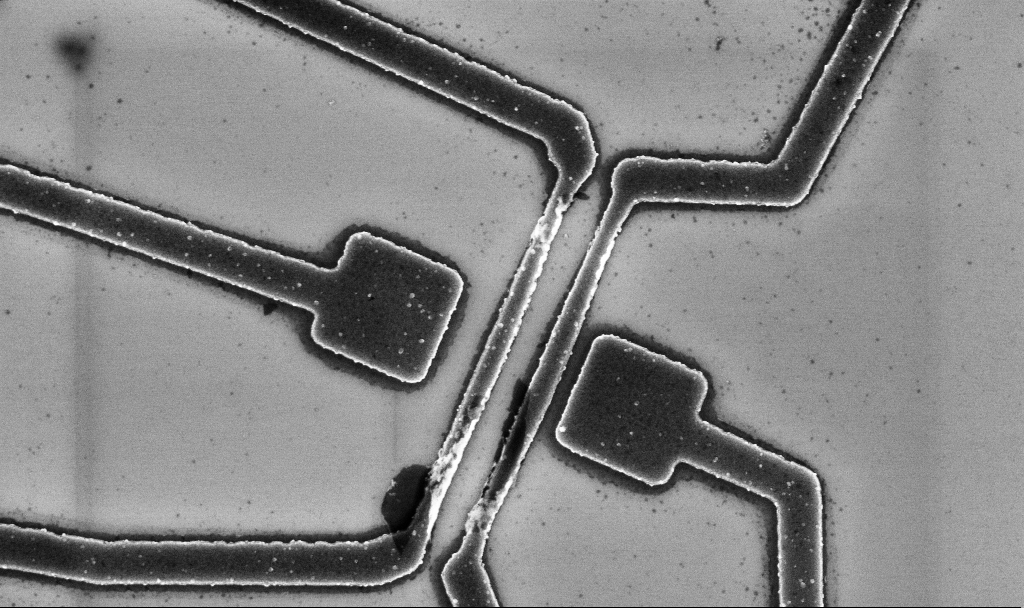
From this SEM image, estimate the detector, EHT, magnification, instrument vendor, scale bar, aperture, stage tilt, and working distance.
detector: InLens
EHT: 5 kV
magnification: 20 K X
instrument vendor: Zeiss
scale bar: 2000 nm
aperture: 30 µm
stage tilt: -0°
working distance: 10.7 mm